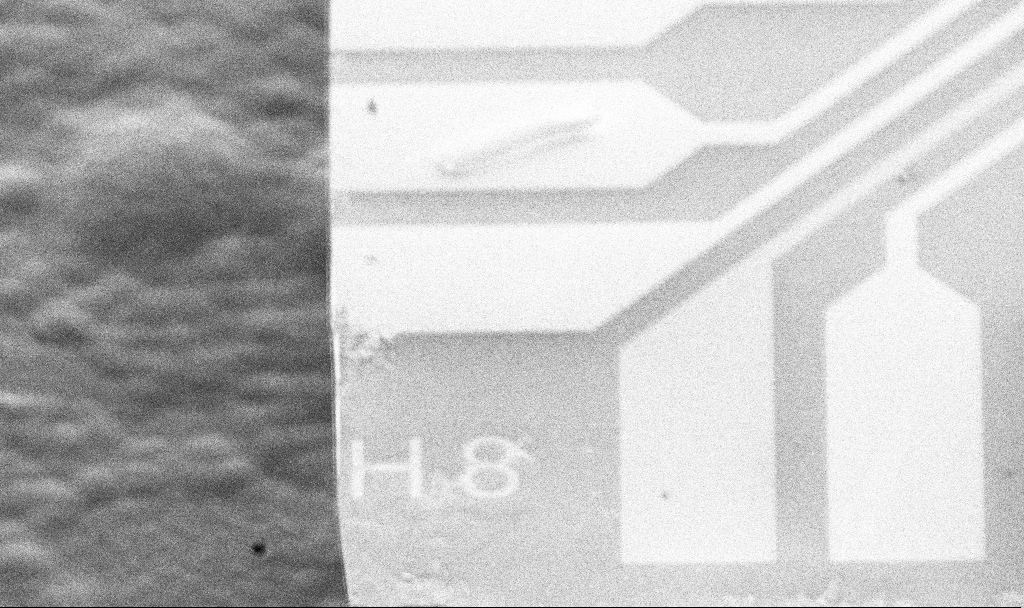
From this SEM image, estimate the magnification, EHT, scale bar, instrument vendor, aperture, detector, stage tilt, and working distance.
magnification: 0.389 K X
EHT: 5 kV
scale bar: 100000 nm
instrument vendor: Zeiss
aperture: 30 µm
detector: SE2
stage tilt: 45°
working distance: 15.6 mm